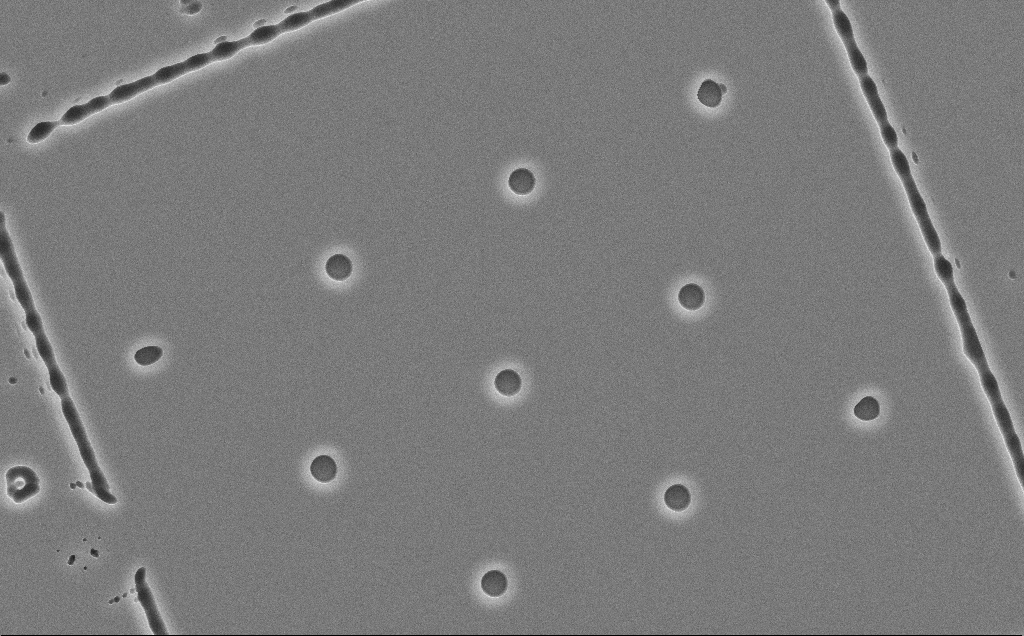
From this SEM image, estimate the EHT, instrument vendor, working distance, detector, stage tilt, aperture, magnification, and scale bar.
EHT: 10 kV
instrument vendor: Zeiss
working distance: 12 mm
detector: SE2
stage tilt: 0°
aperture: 30 µm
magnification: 3.68 K X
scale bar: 10000 nm